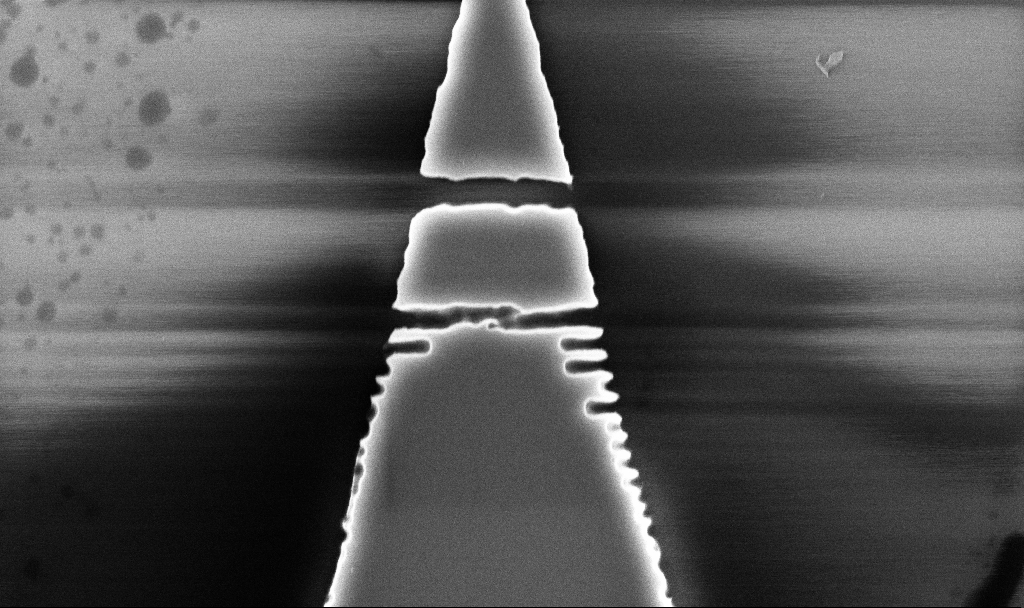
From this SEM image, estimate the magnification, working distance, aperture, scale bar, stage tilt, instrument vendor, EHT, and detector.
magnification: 42.2 K X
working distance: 5.2 mm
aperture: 30 µm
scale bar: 1000 nm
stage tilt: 0°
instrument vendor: Zeiss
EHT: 5 kV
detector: InLens